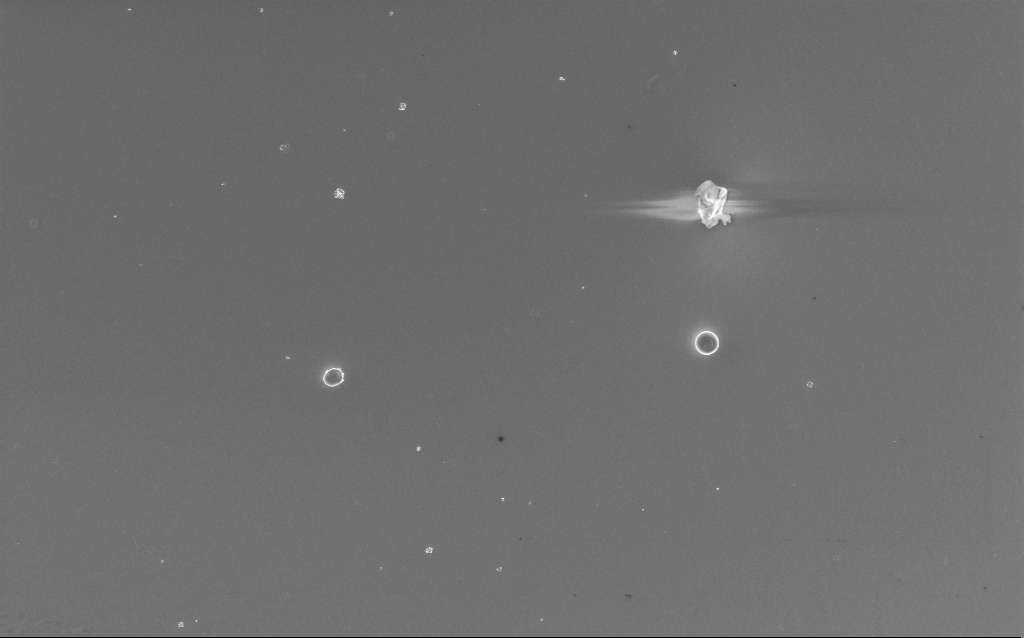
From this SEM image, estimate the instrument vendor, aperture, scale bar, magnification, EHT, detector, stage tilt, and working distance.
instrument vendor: Zeiss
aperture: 30 µm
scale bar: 10000 nm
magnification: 2.32 K X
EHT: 10 kV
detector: InLens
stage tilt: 0°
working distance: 2 mm